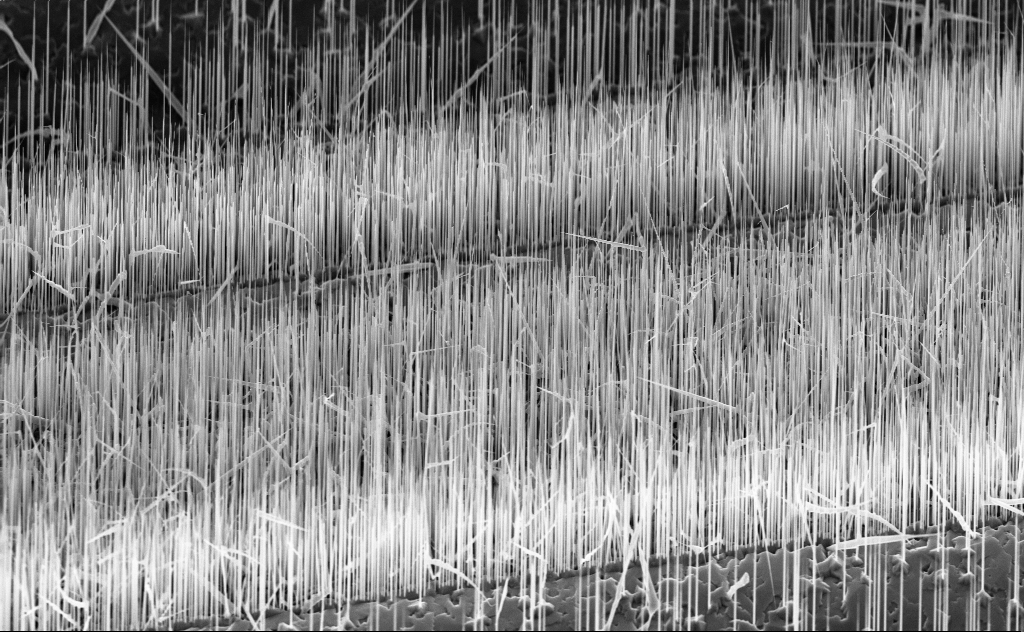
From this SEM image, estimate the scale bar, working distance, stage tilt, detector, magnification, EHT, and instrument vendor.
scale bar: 10000 nm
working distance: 6 mm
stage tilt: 45°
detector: InLens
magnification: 5 K X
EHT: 10 kV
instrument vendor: Zeiss